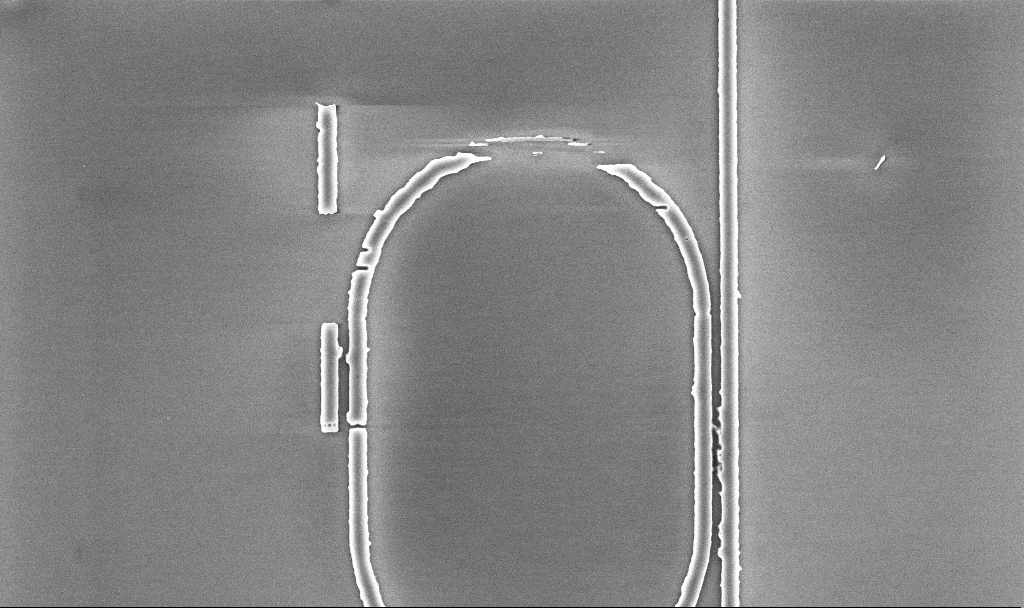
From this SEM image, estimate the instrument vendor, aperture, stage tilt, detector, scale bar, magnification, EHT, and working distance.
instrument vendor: Zeiss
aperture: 30 µm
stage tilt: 0°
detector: InLens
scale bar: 2000 nm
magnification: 13.49 K X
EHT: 5 kV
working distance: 5.2 mm